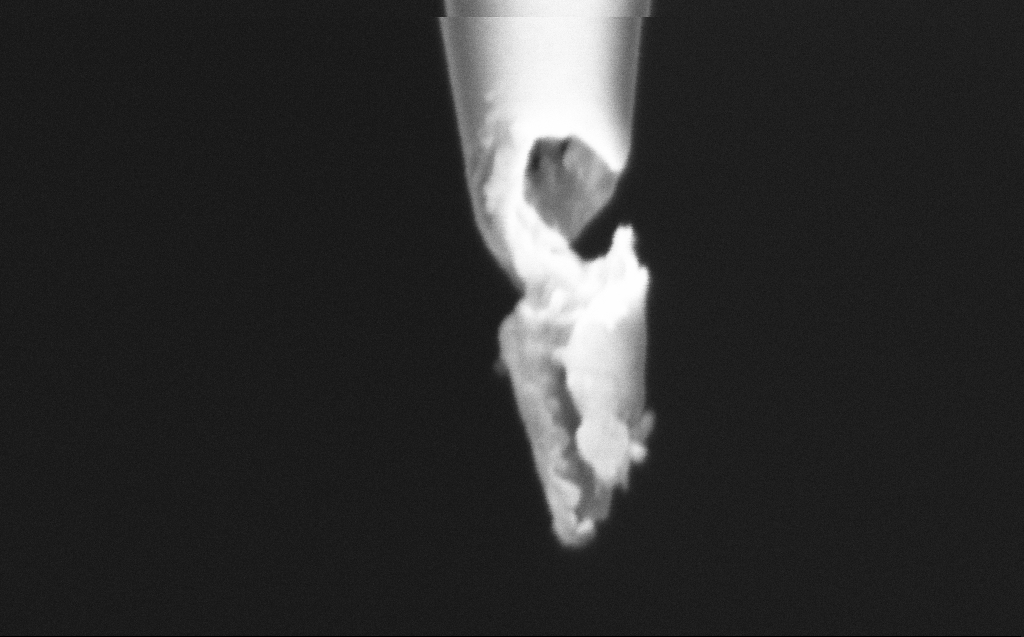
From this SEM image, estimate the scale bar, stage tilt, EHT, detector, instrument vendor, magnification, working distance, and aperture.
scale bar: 200 nm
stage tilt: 45°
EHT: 2 kV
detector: InLens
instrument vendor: Zeiss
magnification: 250 K X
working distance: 6 mm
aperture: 30 µm